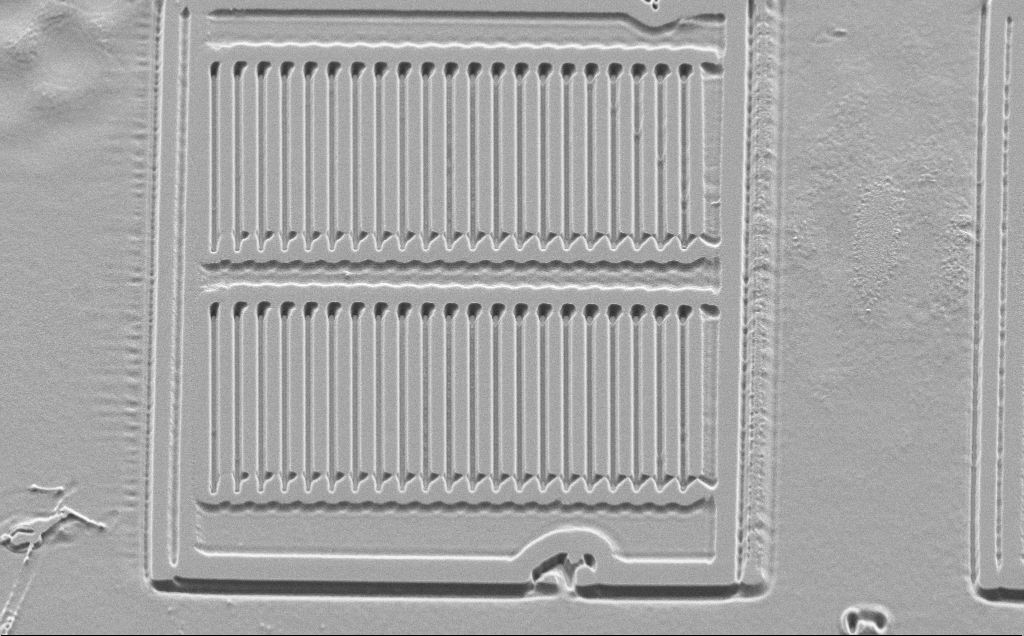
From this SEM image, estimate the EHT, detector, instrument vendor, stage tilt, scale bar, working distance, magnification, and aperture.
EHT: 5 kV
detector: SE2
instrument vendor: Zeiss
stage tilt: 45°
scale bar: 10000 nm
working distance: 10 mm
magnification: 2.14 K X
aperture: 30 µm